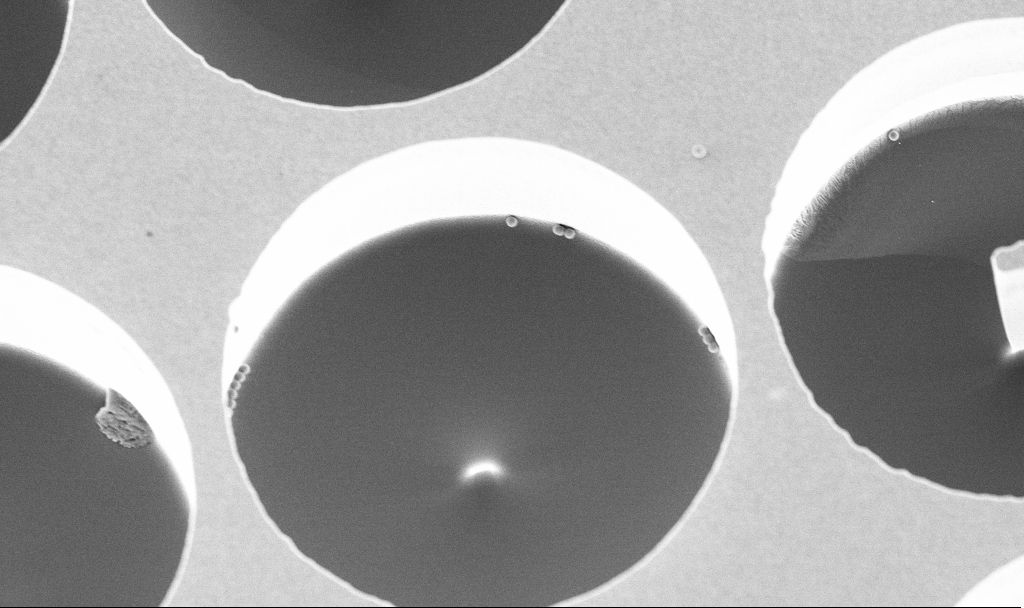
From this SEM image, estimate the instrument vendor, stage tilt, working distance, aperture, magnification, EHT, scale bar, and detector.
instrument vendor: Zeiss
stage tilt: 20°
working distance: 3.6 mm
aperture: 30 µm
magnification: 6.22 K X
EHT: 3 kV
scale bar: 10000 nm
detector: InLens